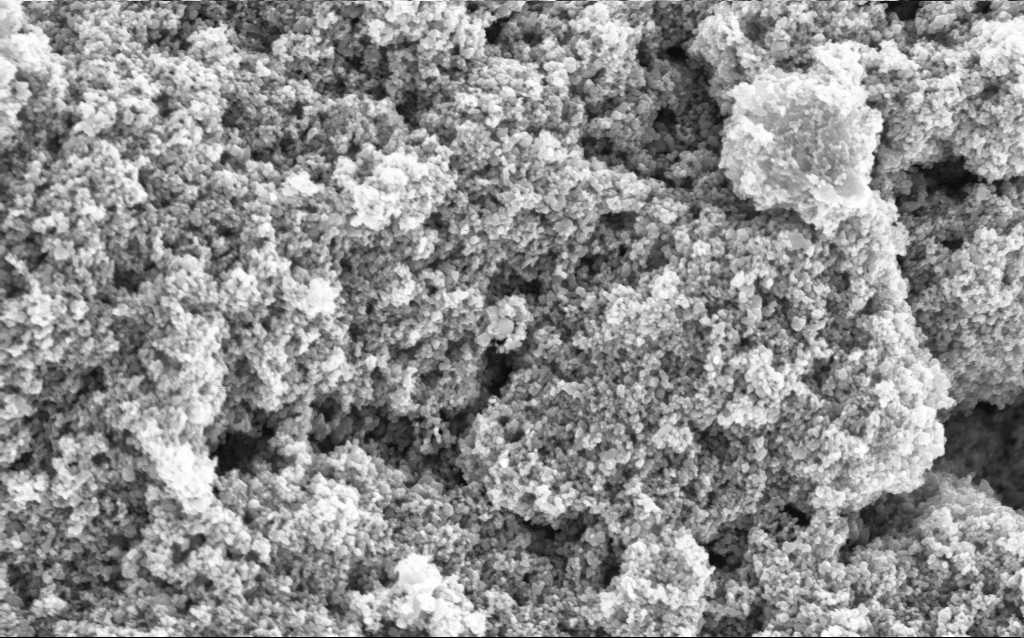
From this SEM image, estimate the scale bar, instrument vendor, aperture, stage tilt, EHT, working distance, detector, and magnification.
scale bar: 1000 nm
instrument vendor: Zeiss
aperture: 30 µm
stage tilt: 0°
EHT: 5 kV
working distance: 4.7 mm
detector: InLens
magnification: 68.65 K X